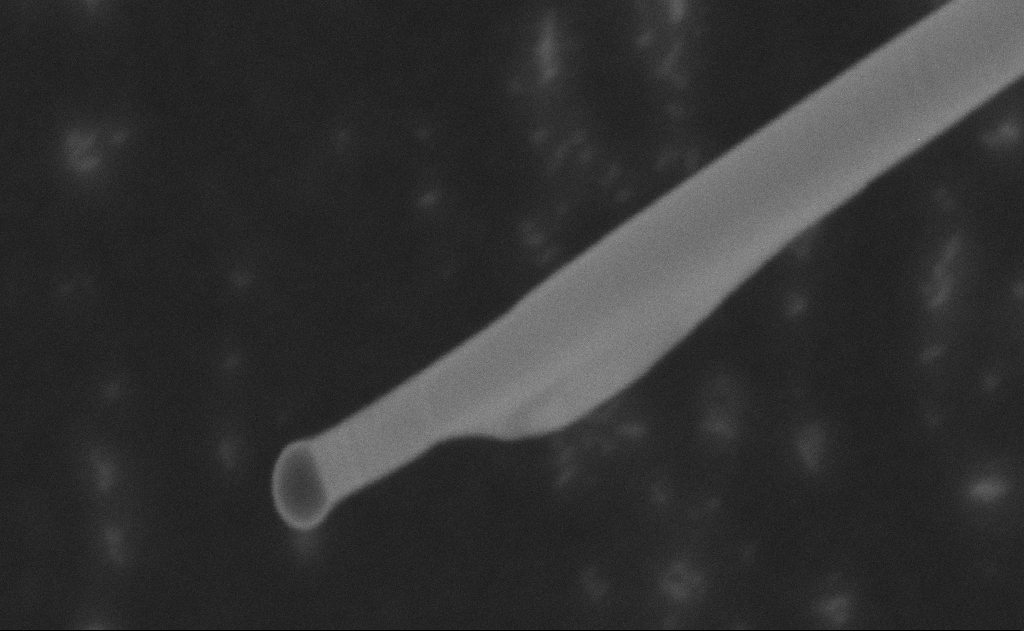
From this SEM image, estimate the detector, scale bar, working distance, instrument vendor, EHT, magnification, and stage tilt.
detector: SE2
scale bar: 100 nm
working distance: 9 mm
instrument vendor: Zeiss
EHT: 20 kV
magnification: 424.86 K X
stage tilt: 0°